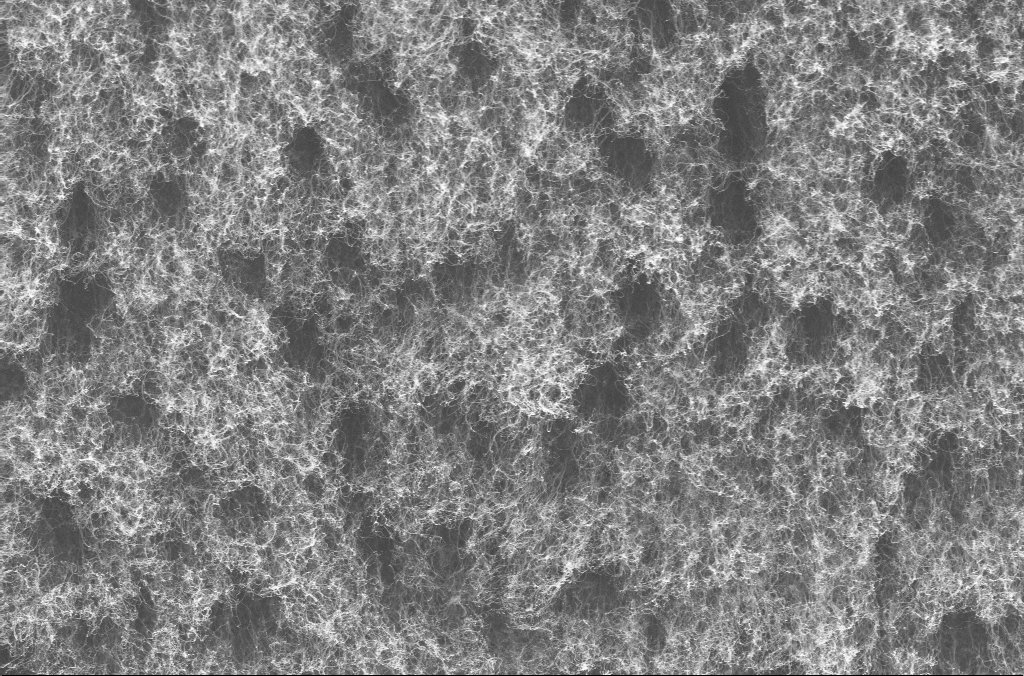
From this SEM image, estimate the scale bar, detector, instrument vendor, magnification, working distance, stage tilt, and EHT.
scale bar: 1000 nm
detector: InLens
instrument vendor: Zeiss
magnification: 20 K X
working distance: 3.9 mm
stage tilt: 0°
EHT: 10 kV